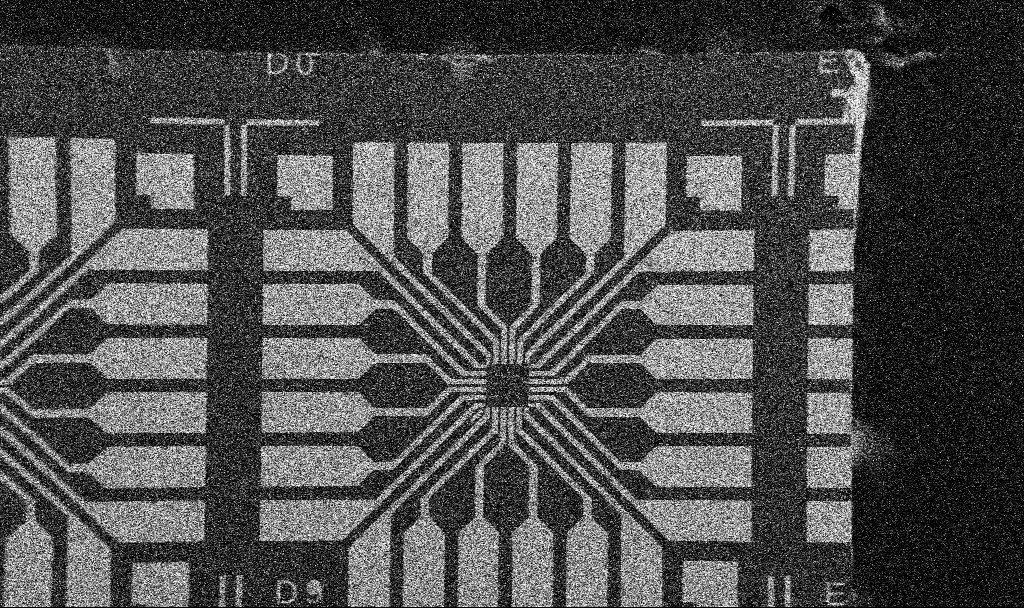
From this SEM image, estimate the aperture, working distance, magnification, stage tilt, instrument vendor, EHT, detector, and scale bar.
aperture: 30 µm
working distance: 10.7 mm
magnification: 0.1 K X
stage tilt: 0°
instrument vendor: Zeiss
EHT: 5 kV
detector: SE2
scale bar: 200000 nm